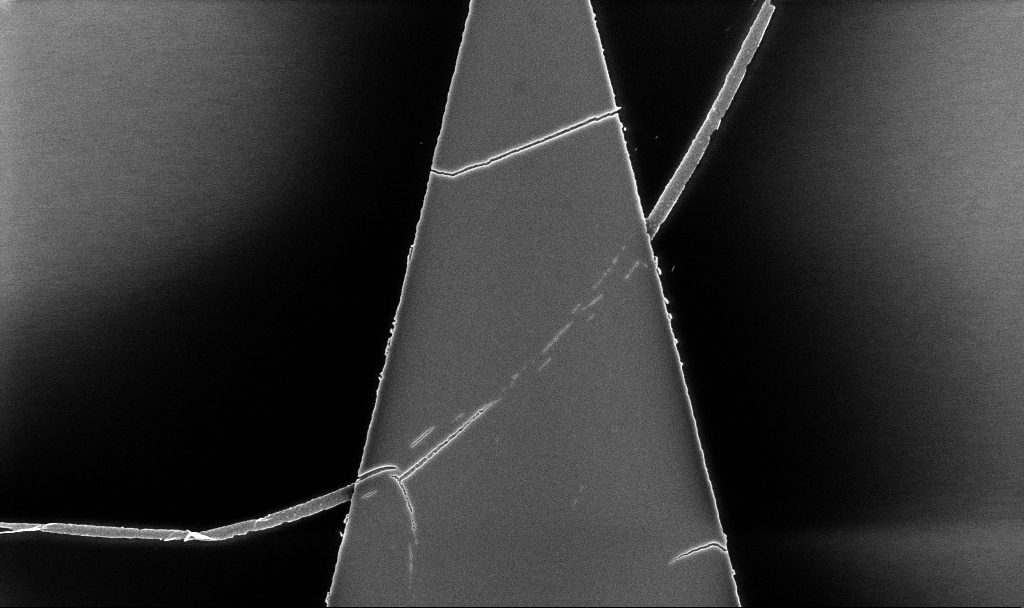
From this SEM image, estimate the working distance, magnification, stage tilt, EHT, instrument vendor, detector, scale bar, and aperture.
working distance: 5.2 mm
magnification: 8.51 K X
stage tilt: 0°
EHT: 5 kV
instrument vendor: Zeiss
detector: InLens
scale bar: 2000 nm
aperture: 30 µm